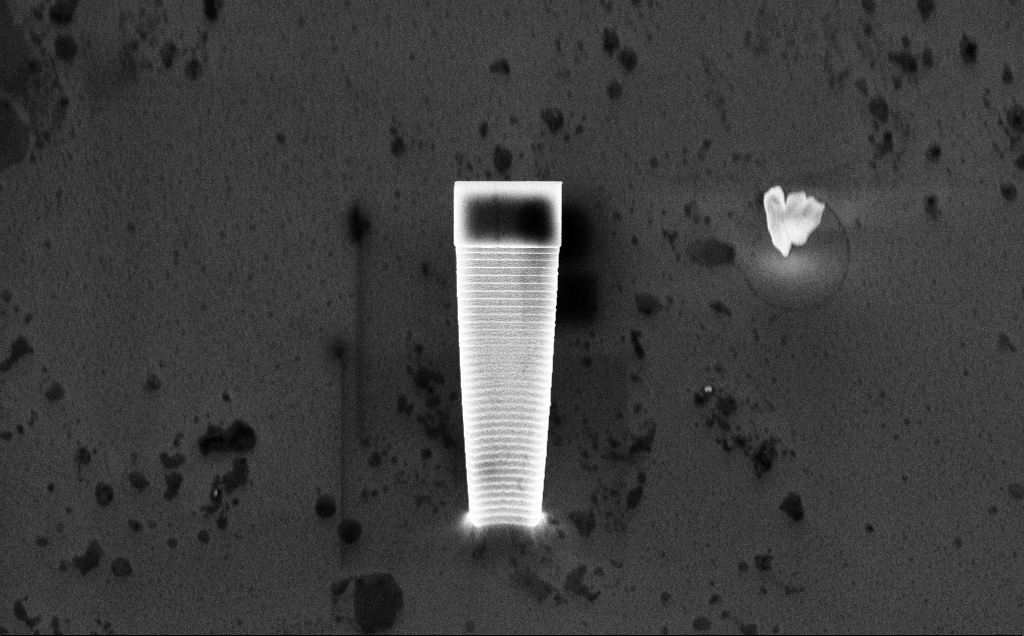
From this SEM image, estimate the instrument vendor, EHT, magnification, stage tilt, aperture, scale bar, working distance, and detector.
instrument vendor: Zeiss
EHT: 10 kV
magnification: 8.12 K X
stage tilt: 45°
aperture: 30 µm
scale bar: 2000 nm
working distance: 5 mm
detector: InLens